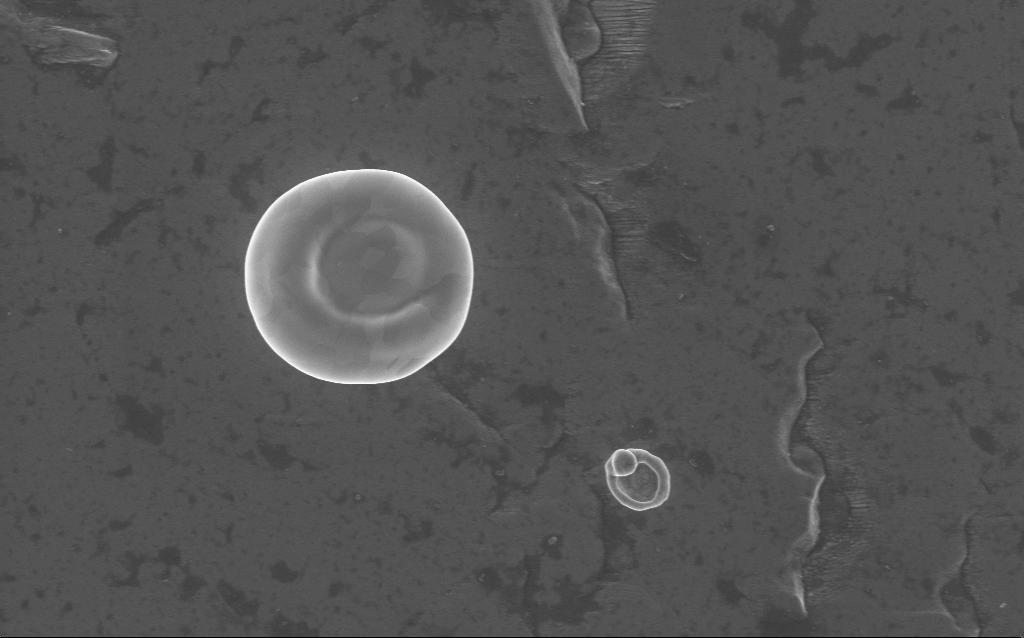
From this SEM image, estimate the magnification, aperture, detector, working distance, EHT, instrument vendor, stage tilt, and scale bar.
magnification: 24.73 K X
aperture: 30 µm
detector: InLens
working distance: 4 mm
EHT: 5 kV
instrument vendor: Zeiss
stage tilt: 0°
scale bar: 2000 nm